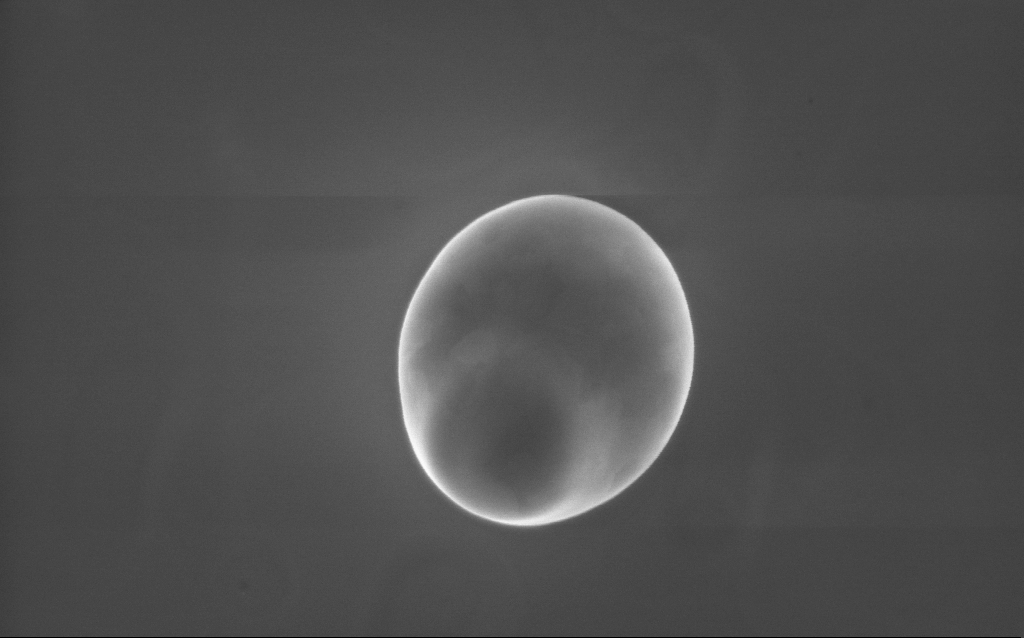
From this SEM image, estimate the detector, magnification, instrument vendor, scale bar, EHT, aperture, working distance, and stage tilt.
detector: InLens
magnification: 42 K X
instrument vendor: Zeiss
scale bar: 1000 nm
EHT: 10 kV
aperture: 30 µm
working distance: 3 mm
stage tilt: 0°